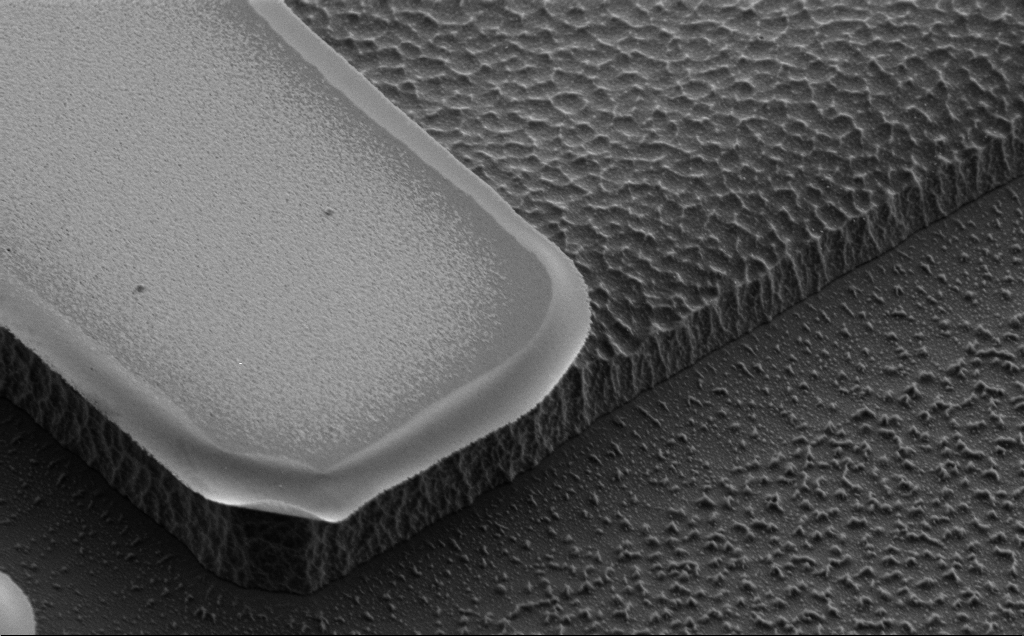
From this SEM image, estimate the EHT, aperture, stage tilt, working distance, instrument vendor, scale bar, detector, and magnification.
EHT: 2 kV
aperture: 30 µm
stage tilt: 43°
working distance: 10 mm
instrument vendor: Zeiss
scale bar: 1000 nm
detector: SE2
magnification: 19.73 K X